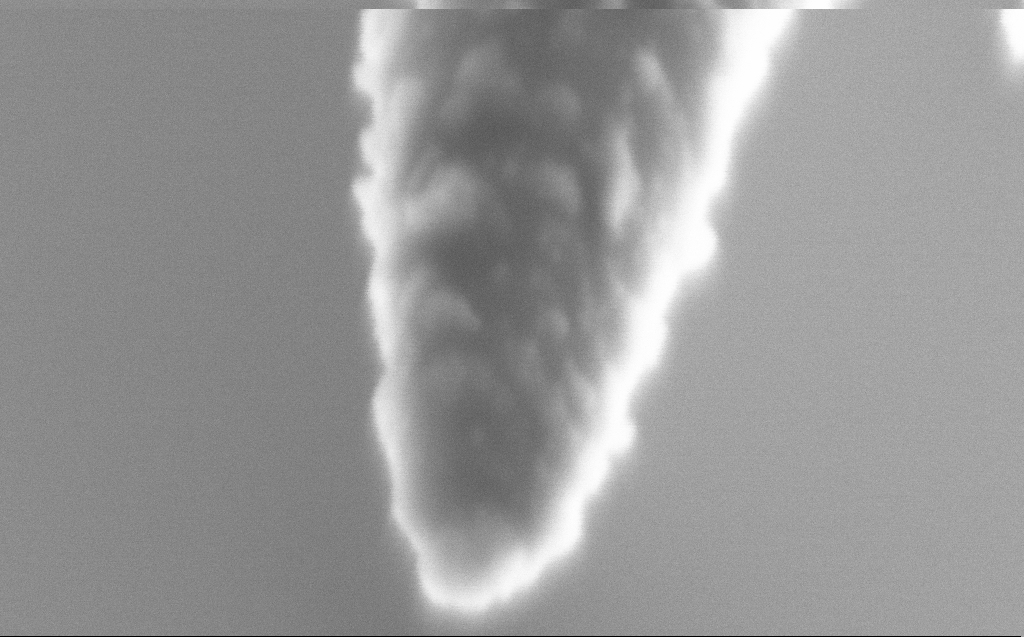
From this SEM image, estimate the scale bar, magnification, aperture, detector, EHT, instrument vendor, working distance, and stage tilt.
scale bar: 100 nm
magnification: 400 K X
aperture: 30 µm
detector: SE2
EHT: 2 kV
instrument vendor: Zeiss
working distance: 6 mm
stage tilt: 45°